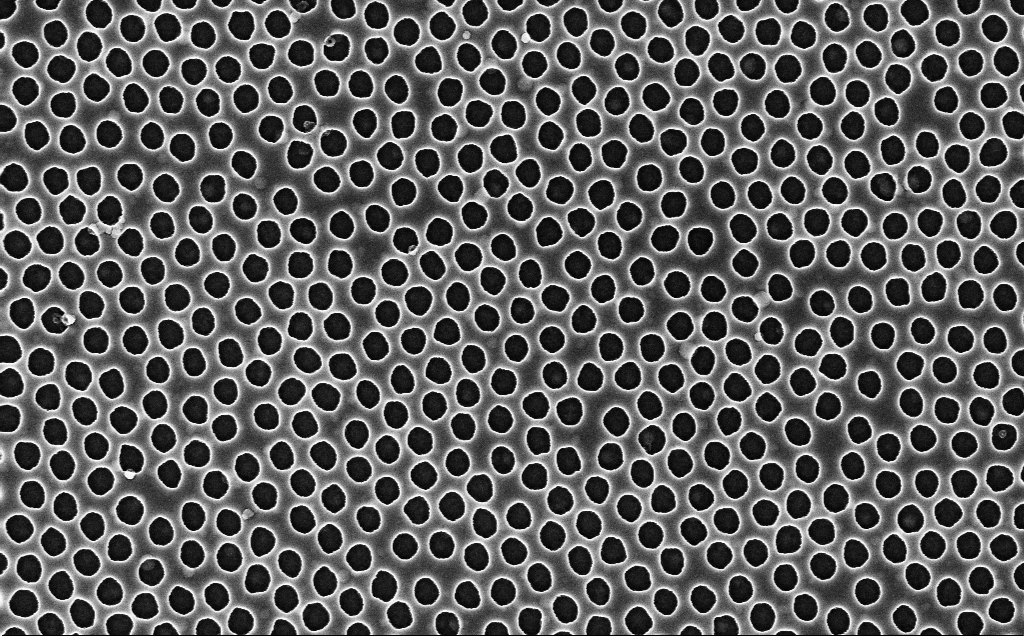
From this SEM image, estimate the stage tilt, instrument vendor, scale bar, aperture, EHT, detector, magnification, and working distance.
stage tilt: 0°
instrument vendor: Zeiss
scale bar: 1000 nm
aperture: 30 µm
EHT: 3 kV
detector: InLens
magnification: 40 K X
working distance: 2.5 mm